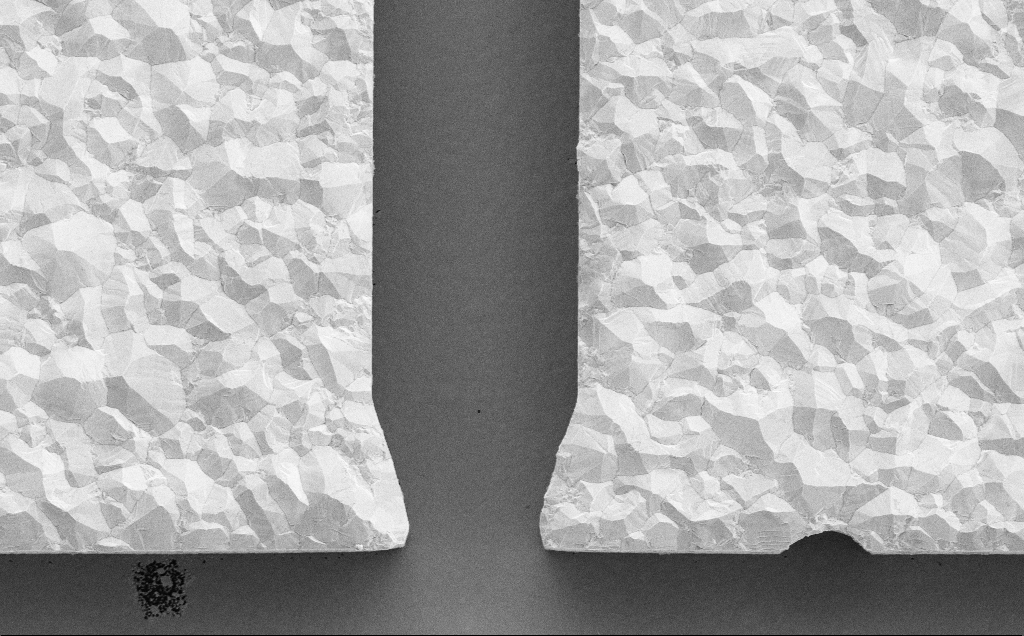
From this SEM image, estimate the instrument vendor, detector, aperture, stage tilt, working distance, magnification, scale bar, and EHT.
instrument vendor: Zeiss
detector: SE2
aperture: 30 µm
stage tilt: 0°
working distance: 13 mm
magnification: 7.35 K X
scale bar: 2000 nm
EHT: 5 kV